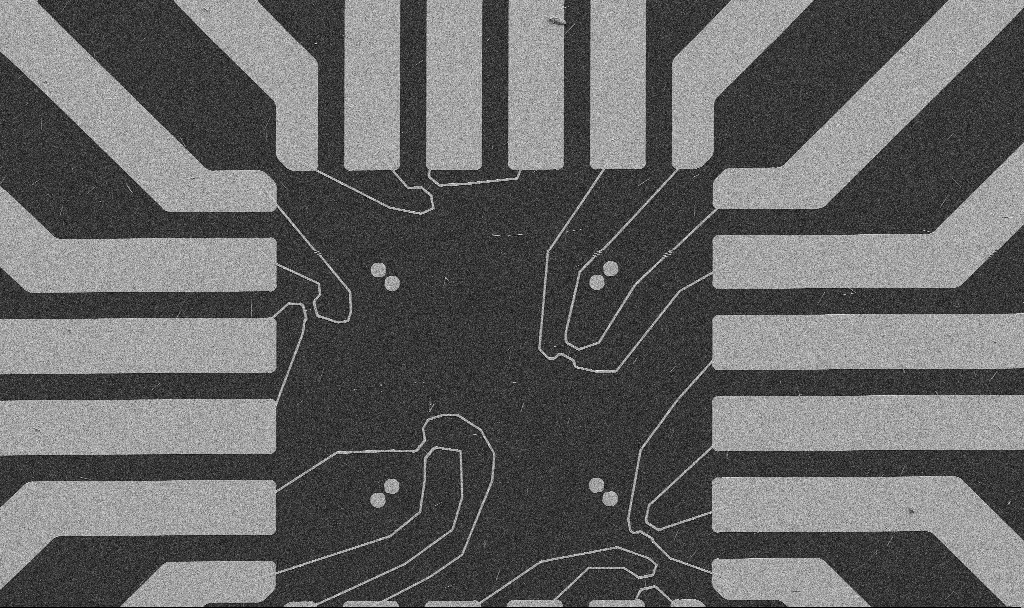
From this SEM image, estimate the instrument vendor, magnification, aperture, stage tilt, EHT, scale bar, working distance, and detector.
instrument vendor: Zeiss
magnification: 1 K X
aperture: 30 µm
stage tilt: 0°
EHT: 5 kV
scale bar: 20000 nm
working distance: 10.7 mm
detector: SE2